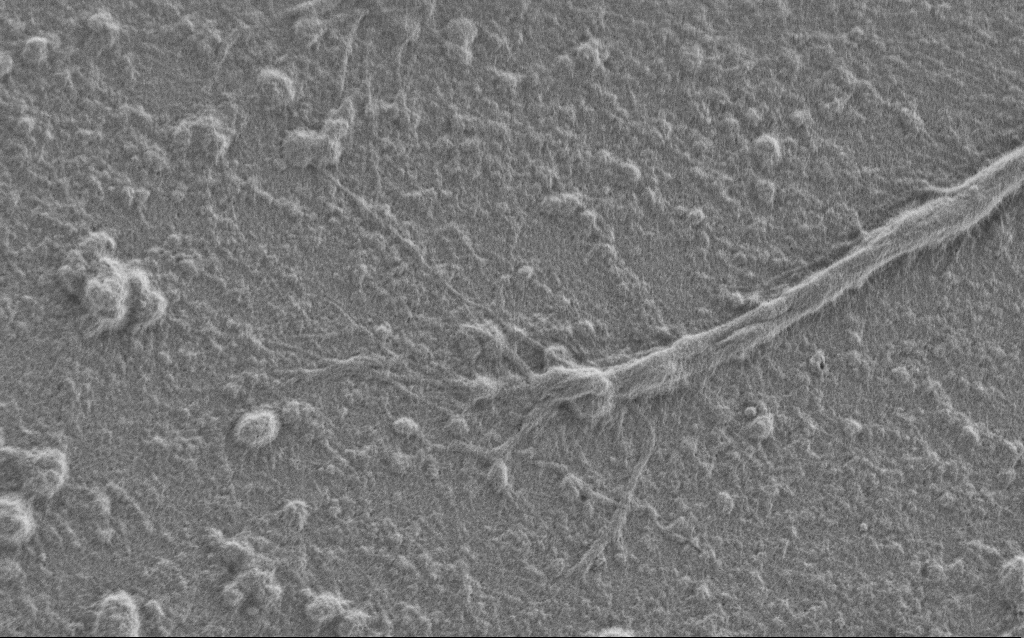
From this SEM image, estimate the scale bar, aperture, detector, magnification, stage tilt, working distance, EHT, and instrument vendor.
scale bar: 2000 nm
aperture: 30 µm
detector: SE2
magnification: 7.5 K X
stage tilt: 0°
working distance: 6 mm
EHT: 1 kV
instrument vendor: Zeiss